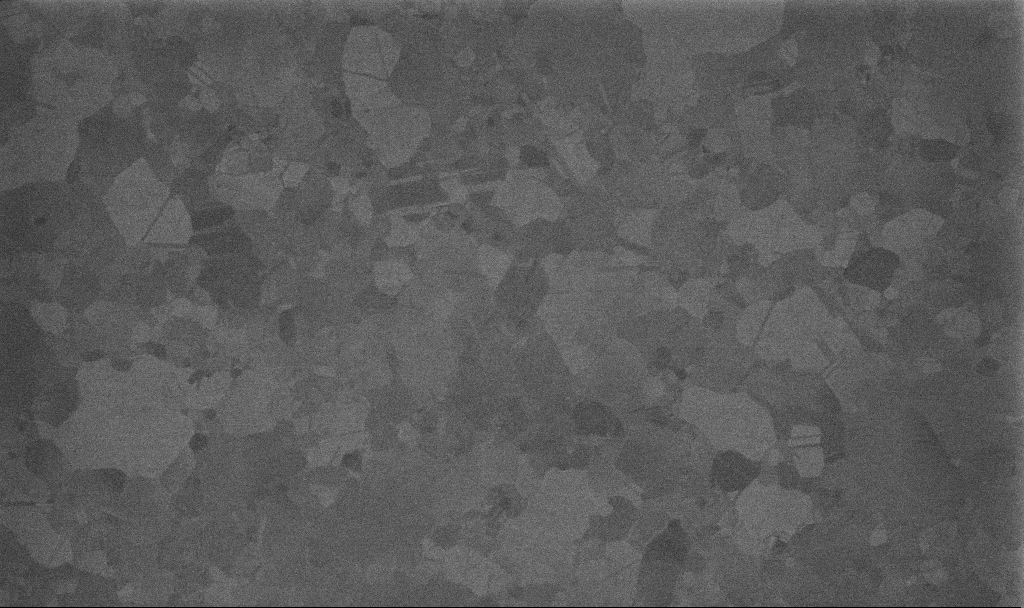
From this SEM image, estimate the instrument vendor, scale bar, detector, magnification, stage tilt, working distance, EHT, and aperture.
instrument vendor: Zeiss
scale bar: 200 nm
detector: InLens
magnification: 100 K X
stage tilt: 0°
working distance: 3.4 mm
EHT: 10 kV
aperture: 30 µm